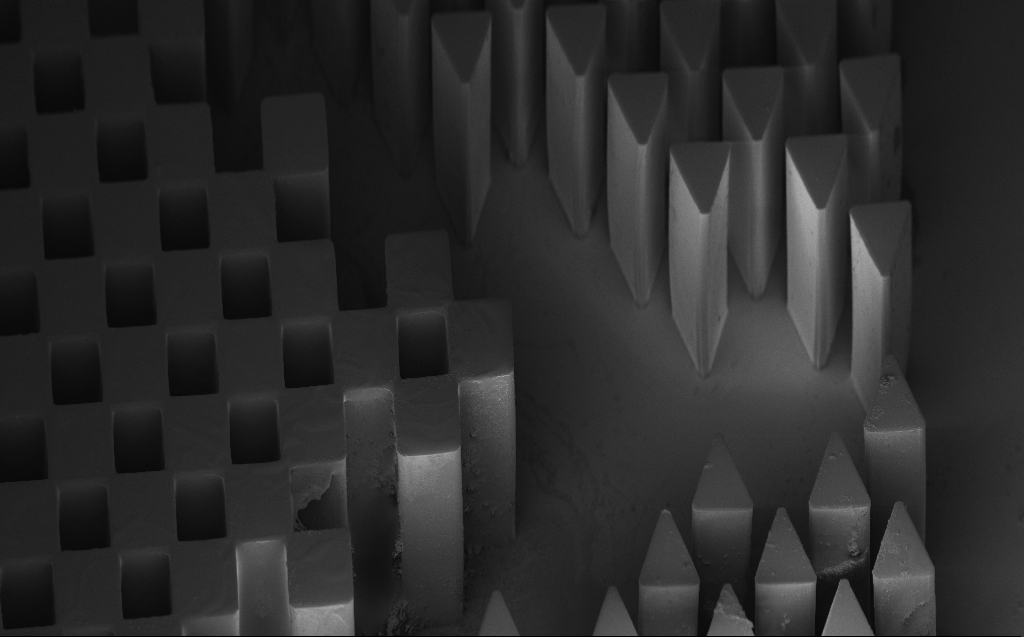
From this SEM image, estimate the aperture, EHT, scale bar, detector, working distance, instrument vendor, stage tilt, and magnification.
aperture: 30 µm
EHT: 3 kV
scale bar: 200000 nm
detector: InLens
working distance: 6 mm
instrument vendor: Zeiss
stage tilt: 45°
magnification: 0.252 K X